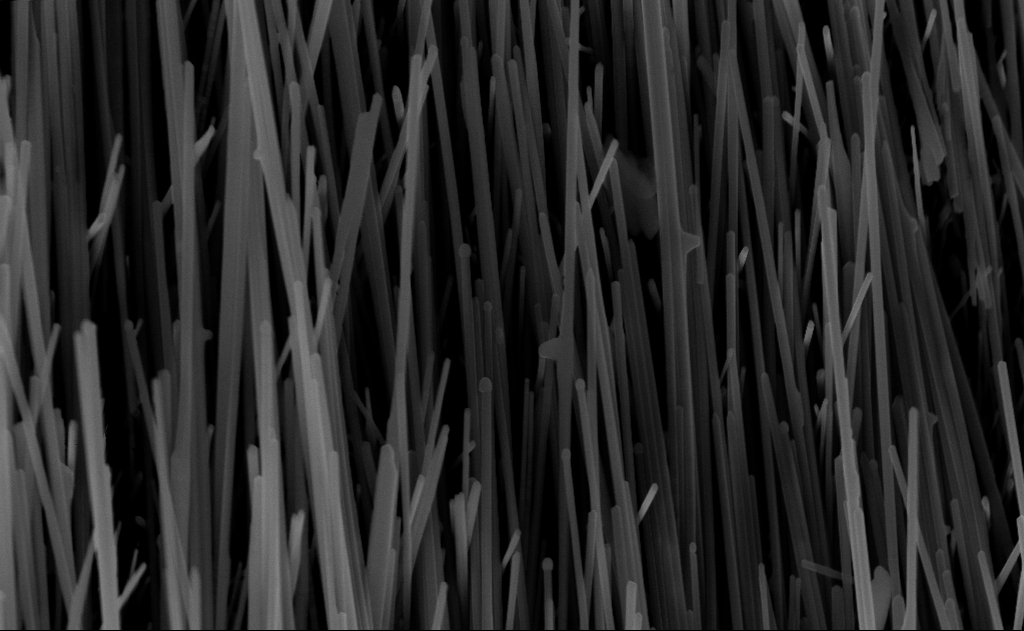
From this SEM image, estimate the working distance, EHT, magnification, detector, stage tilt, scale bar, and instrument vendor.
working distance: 7 mm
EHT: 10 kV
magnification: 40 K X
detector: InLens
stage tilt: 0°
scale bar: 1000 nm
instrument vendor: Zeiss